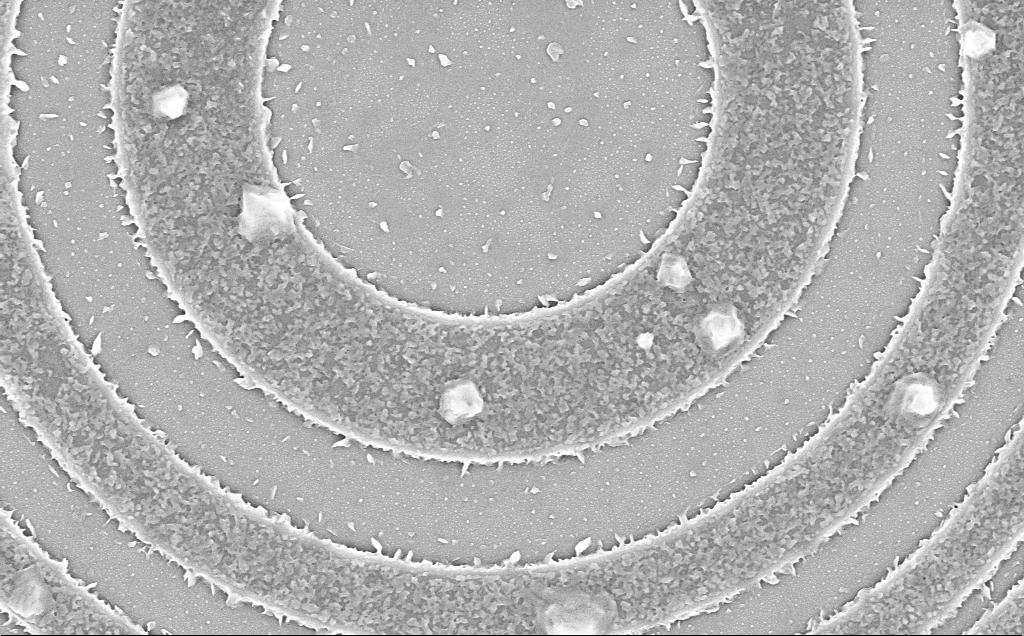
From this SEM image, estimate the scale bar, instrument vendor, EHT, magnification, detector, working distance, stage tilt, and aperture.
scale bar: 2000 nm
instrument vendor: Zeiss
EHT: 5 kV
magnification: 22.57 K X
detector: InLens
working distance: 6 mm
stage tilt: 0°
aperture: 30 µm